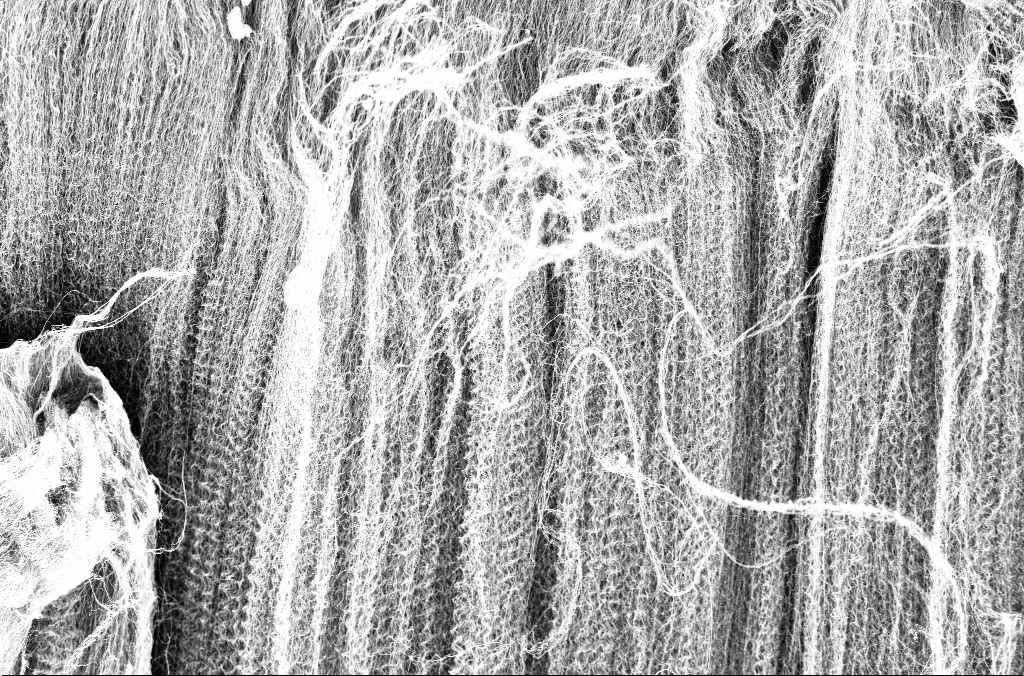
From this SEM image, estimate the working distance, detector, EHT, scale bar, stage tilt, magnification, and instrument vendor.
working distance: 3.3 mm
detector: InLens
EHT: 3 kV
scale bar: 10000 nm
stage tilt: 45°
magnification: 5 K X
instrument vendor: Zeiss